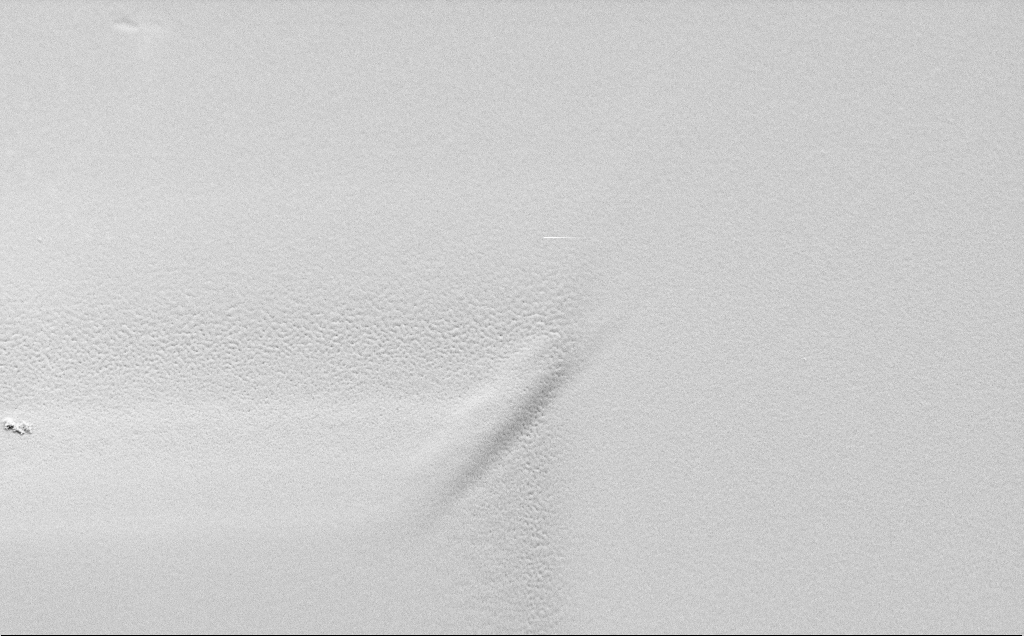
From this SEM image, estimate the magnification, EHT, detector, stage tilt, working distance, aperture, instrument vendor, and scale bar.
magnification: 10.31 K X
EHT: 1.5 kV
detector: SE2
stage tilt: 45°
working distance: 5 mm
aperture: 30 µm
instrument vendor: Zeiss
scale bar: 2000 nm